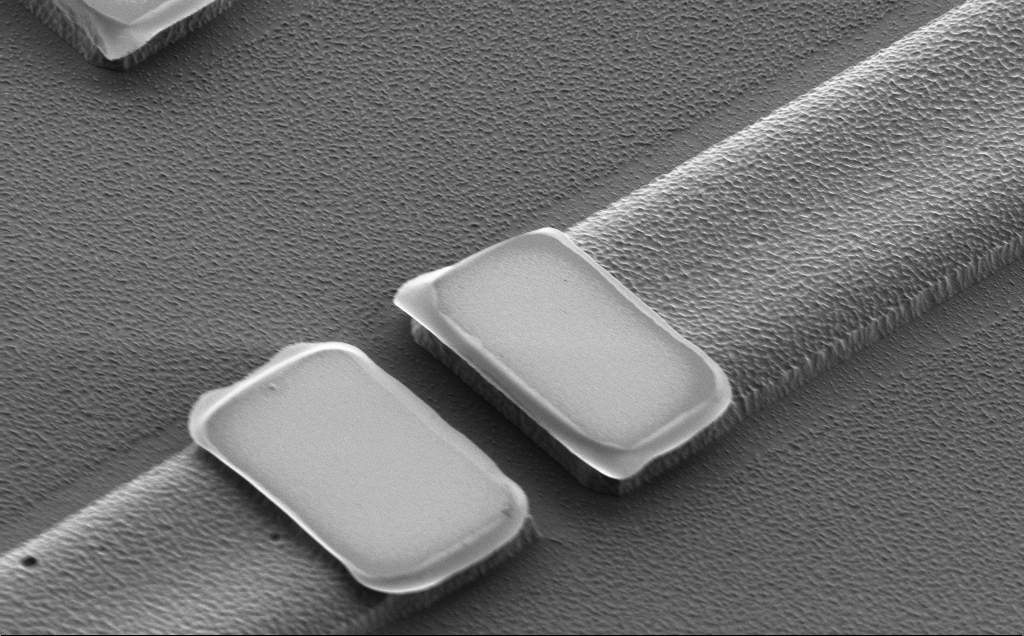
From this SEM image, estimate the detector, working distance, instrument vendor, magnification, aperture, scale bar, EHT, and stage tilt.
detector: SE2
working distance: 10 mm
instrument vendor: Zeiss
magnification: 7.12 K X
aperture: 30 µm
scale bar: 10000 nm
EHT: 2 kV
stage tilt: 43°